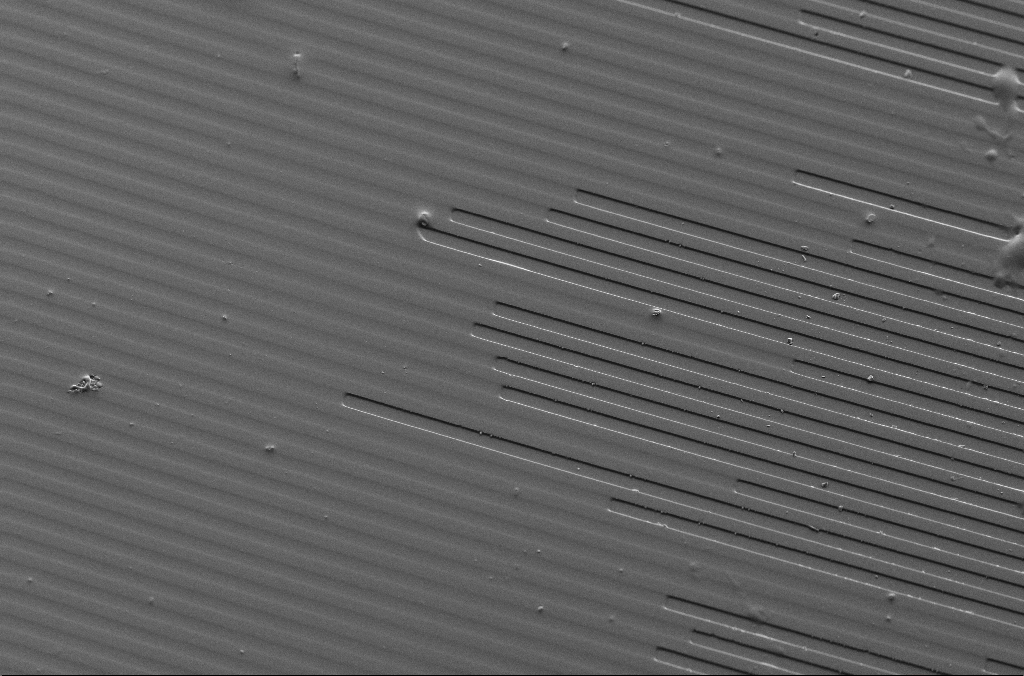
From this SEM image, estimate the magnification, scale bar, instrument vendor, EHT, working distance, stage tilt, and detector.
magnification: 0.5 K X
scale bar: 100000 nm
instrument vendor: Zeiss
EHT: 5 kV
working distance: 7.2 mm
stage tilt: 40°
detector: SE2